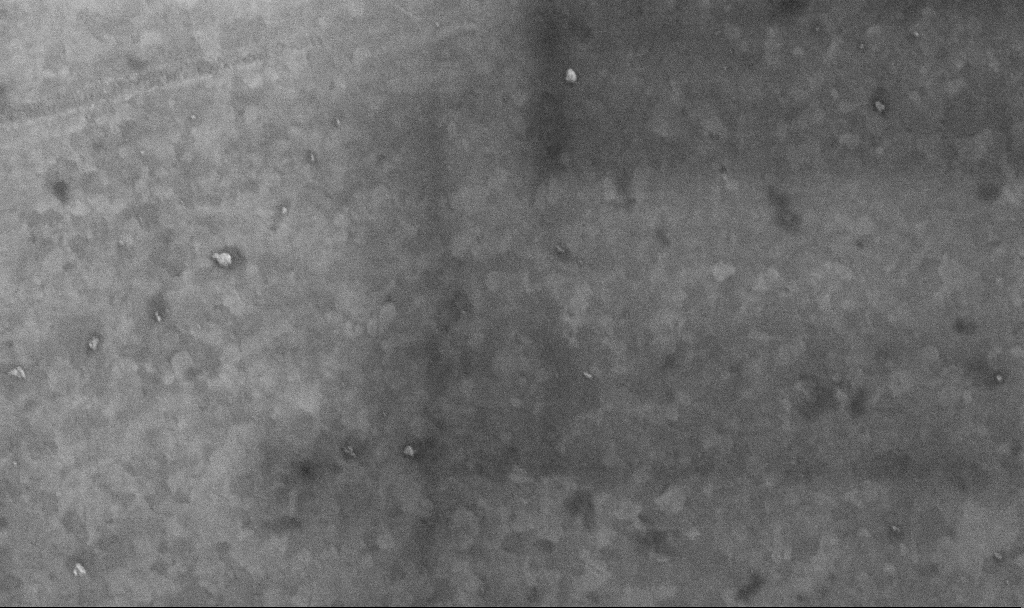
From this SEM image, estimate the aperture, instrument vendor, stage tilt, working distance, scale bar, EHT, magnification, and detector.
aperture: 30 µm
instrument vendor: Zeiss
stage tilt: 0°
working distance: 3.4 mm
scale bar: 200 nm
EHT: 10 kV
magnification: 100.94 K X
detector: InLens